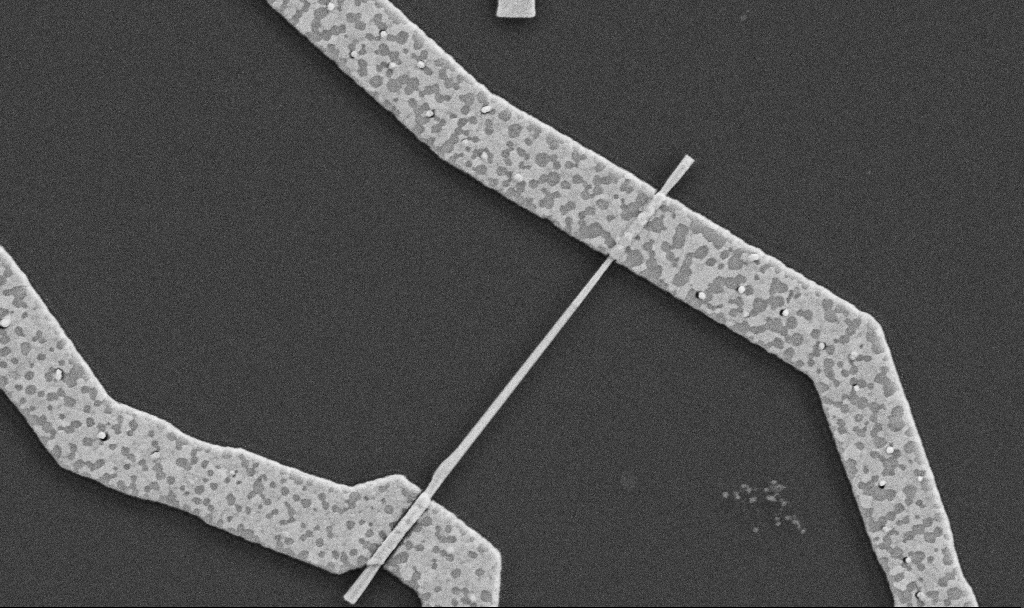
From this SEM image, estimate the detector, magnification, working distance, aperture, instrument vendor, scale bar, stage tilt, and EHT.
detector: SE2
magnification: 30 K X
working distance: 8.7 mm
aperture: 30 µm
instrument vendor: Zeiss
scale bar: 1000 nm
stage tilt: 0°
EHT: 5 kV